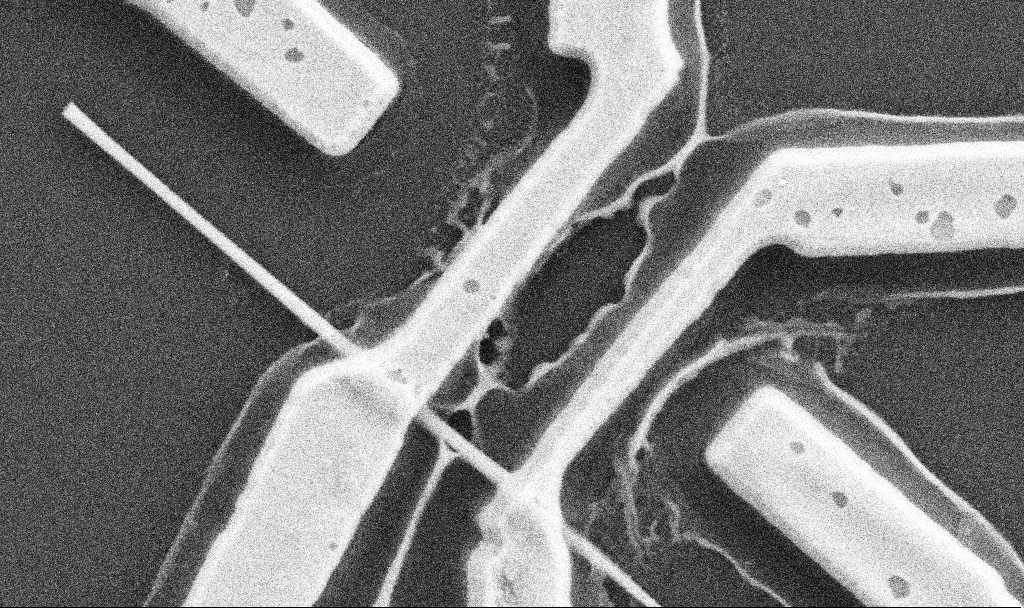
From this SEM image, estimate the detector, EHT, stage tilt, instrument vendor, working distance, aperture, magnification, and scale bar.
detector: SE2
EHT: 5 kV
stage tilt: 0°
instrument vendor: Zeiss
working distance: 10.7 mm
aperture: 30 µm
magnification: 60 K X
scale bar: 1000 nm